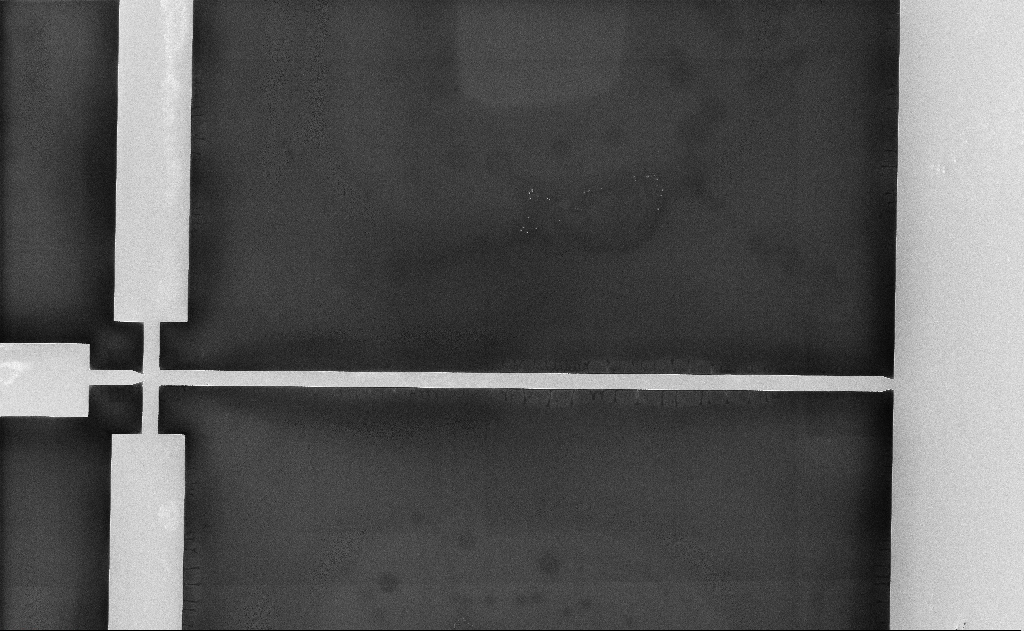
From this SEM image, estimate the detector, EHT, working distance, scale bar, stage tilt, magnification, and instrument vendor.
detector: InLens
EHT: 10 kV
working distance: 12 mm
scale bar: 100000 nm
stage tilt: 0°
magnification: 0.532 K X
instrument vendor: Zeiss